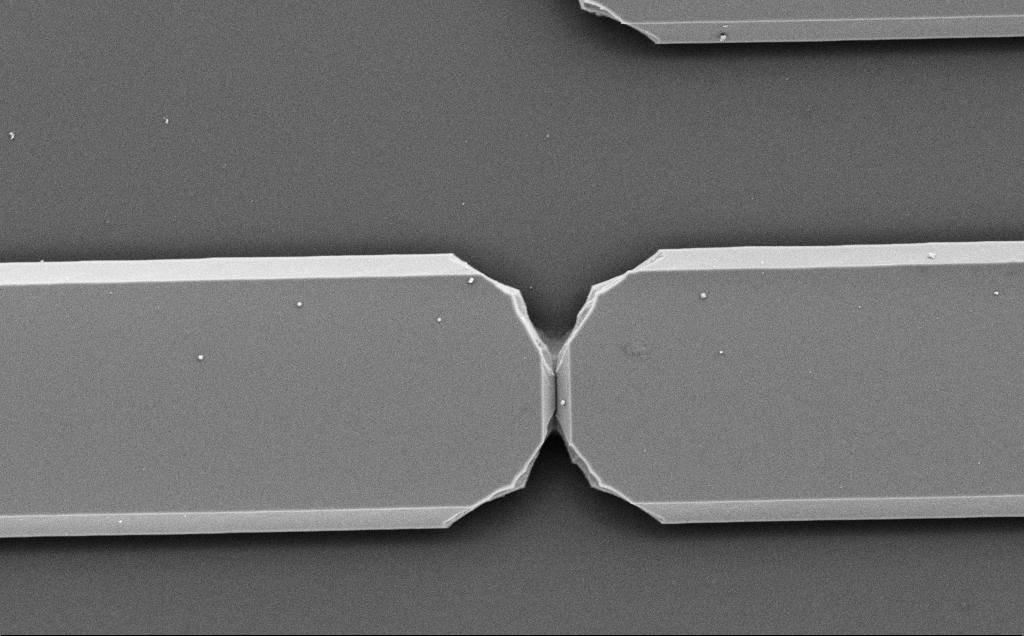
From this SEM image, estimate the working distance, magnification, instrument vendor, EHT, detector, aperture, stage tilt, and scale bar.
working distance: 10 mm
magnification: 4.47 K X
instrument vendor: Zeiss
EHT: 5 kV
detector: SE2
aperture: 30 µm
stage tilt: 0°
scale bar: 10000 nm